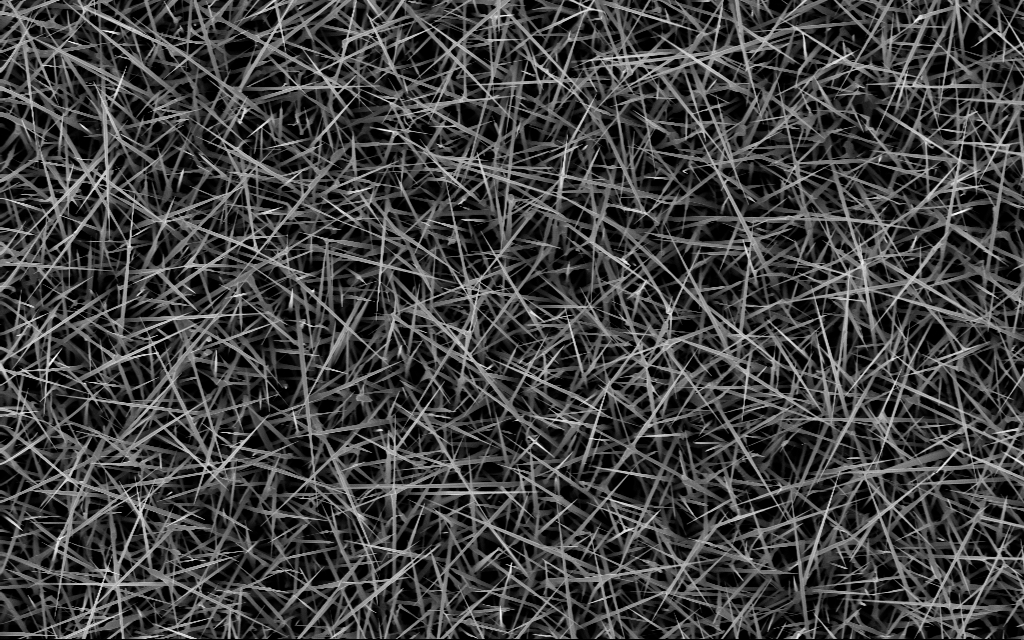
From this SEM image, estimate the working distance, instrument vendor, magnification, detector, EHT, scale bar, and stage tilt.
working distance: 7 mm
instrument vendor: Zeiss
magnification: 10 K X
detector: InLens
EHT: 10 kV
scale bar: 2000 nm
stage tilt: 0°